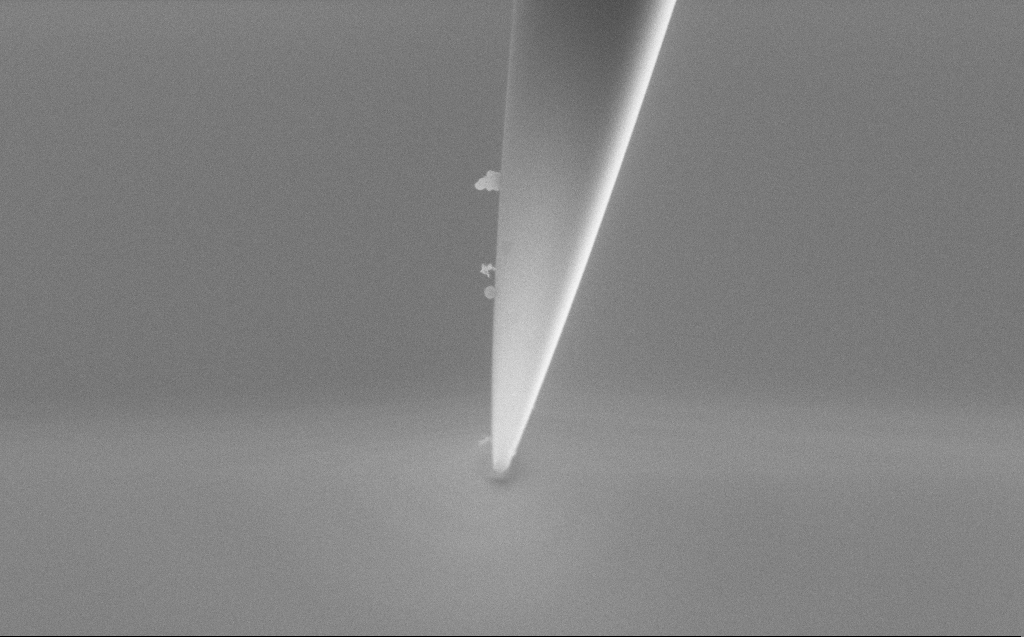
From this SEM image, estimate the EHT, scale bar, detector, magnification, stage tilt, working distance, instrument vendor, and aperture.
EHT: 5 kV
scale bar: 1000 nm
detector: SE2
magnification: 42.22 K X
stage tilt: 45.1°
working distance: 5 mm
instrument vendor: Zeiss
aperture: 30 µm